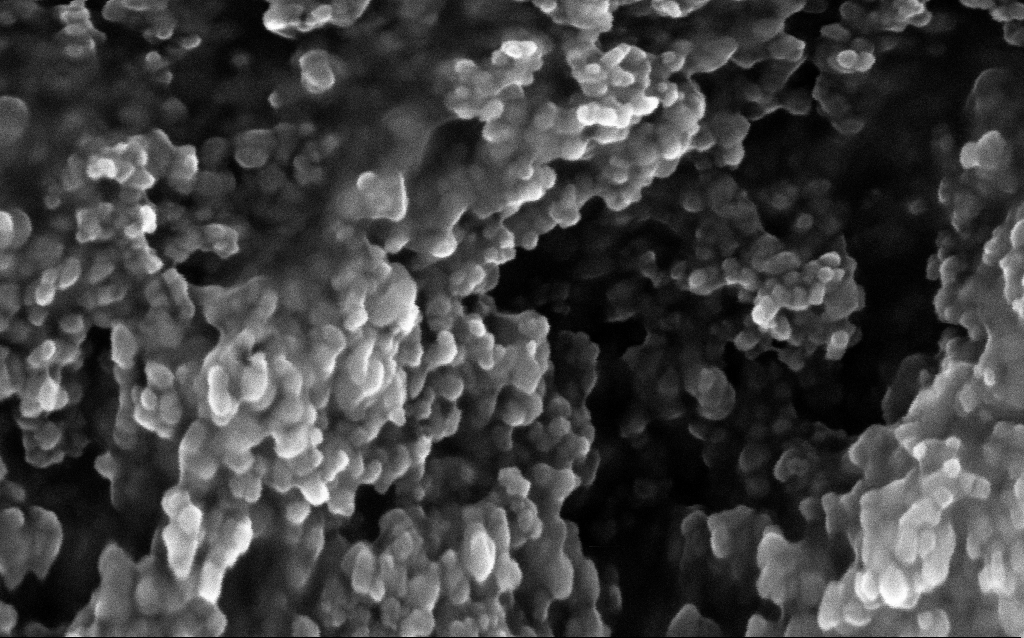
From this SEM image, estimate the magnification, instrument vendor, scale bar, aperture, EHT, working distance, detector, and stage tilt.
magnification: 348.1 K X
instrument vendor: Zeiss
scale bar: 100 nm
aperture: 30 µm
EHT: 10 kV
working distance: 2.6 mm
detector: InLens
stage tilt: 0°